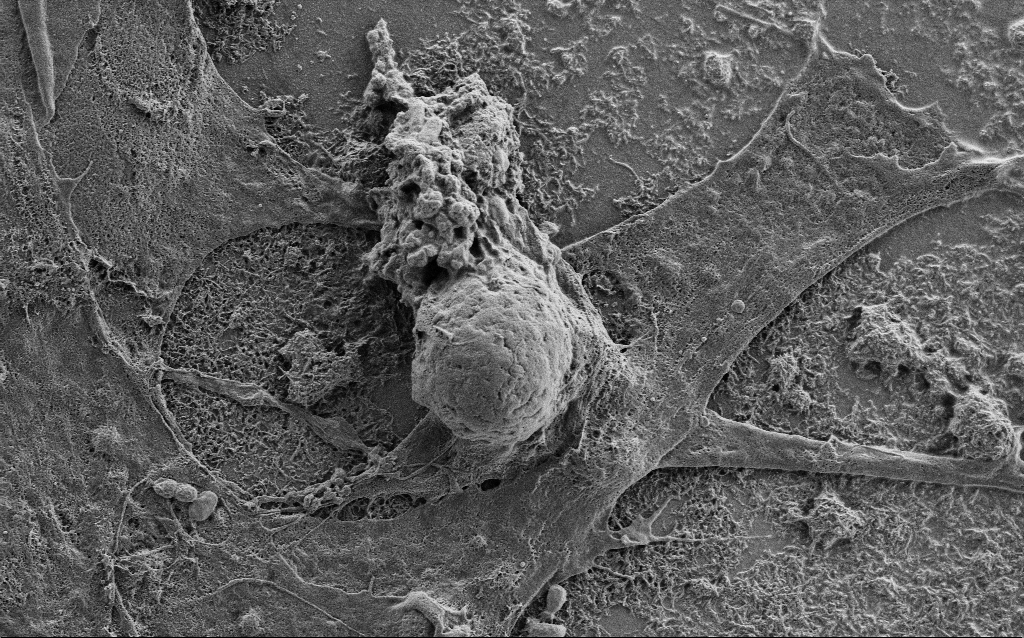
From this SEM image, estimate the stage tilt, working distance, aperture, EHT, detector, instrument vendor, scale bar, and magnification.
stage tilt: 0°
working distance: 6 mm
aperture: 30 µm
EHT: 1 kV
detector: SE2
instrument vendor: Zeiss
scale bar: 2000 nm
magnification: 10 K X